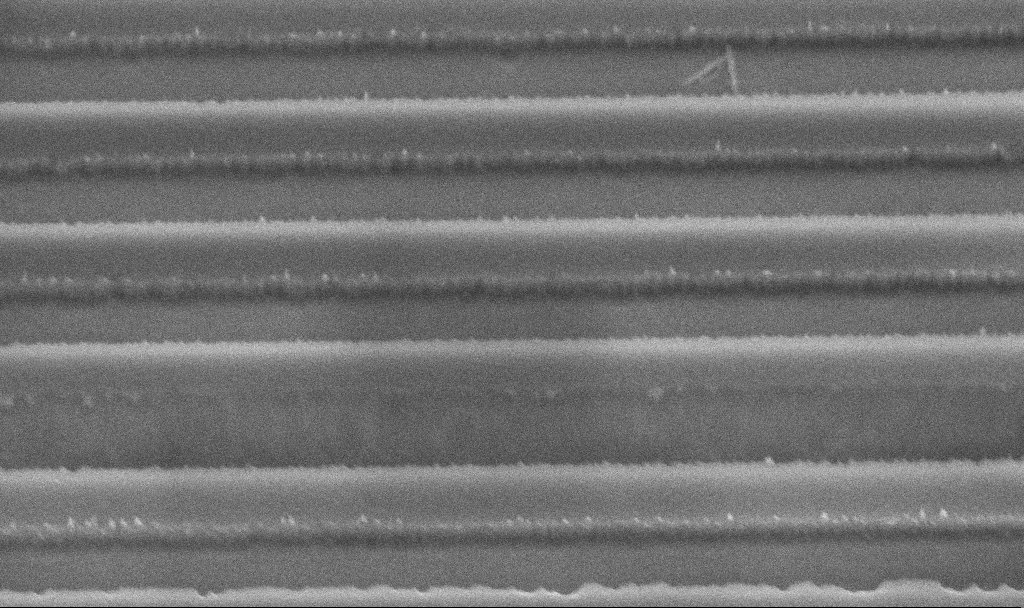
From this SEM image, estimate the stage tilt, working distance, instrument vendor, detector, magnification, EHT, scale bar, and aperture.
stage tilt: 45°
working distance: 9.5 mm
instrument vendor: Zeiss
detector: InLens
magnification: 66.68 K X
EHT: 5 kV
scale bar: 1000 nm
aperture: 30 µm